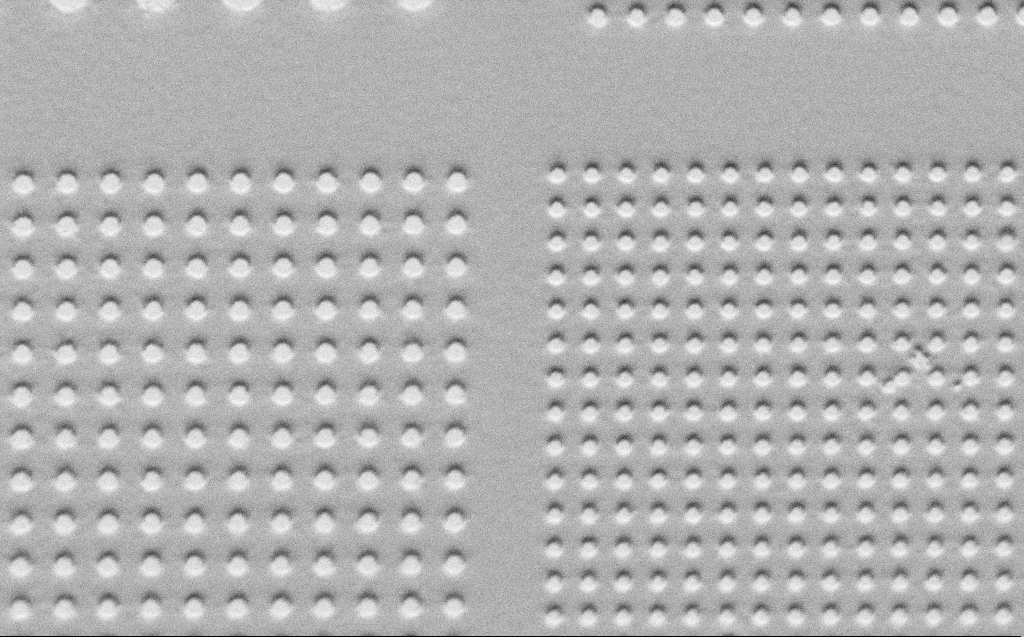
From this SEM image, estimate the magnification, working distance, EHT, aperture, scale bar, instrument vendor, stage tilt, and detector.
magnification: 8.06 K X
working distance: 5 mm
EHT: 1 kV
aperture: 30 µm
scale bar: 2000 nm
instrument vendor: Zeiss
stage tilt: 45°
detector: SE2